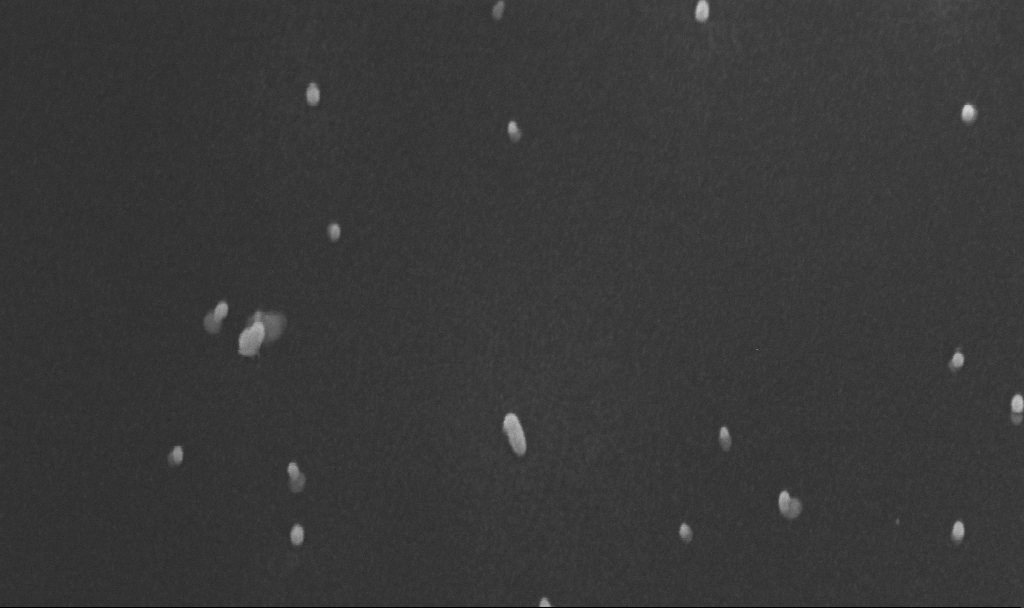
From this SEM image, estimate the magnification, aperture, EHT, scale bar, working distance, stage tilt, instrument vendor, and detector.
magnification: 200 K X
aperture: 30 µm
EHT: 10 kV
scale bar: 100 nm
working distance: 4.8 mm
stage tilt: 45°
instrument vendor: Zeiss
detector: InLens